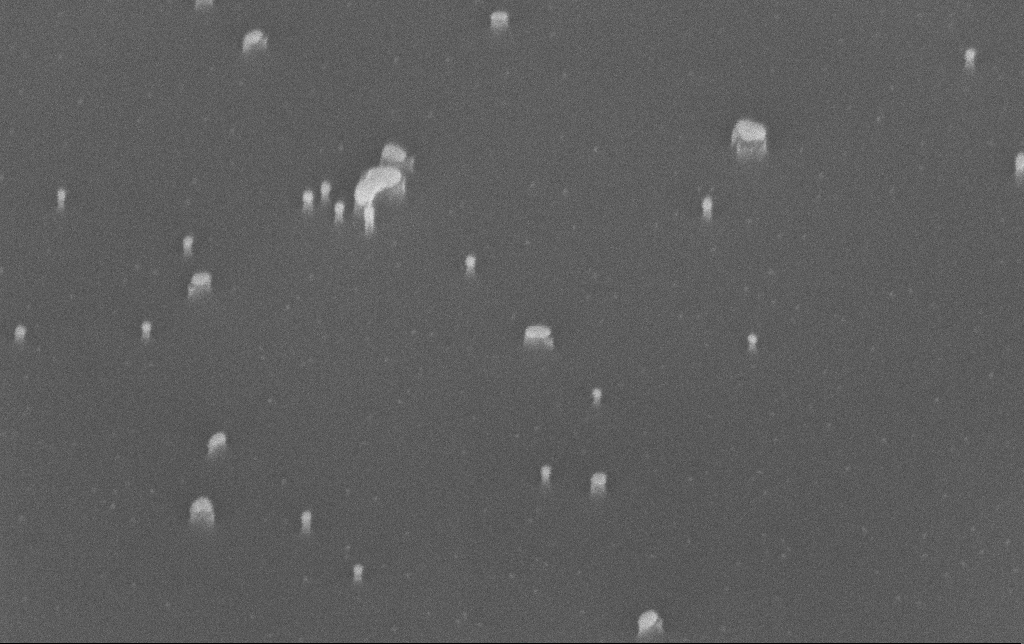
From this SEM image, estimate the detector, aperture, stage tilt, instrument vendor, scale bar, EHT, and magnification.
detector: InLens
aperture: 30 µm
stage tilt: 45°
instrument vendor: Zeiss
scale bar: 100 nm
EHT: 10 kV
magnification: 200 K X